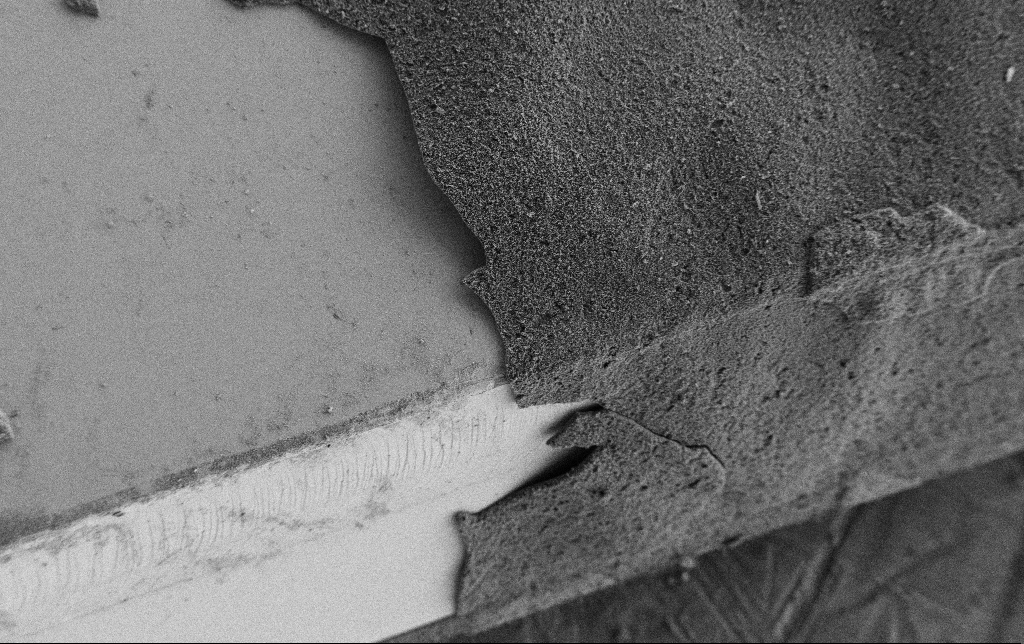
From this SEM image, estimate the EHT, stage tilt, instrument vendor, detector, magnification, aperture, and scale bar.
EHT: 2 kV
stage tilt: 0°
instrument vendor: Zeiss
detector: SE2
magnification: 0.25 K X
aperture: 30 µm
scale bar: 100000 nm